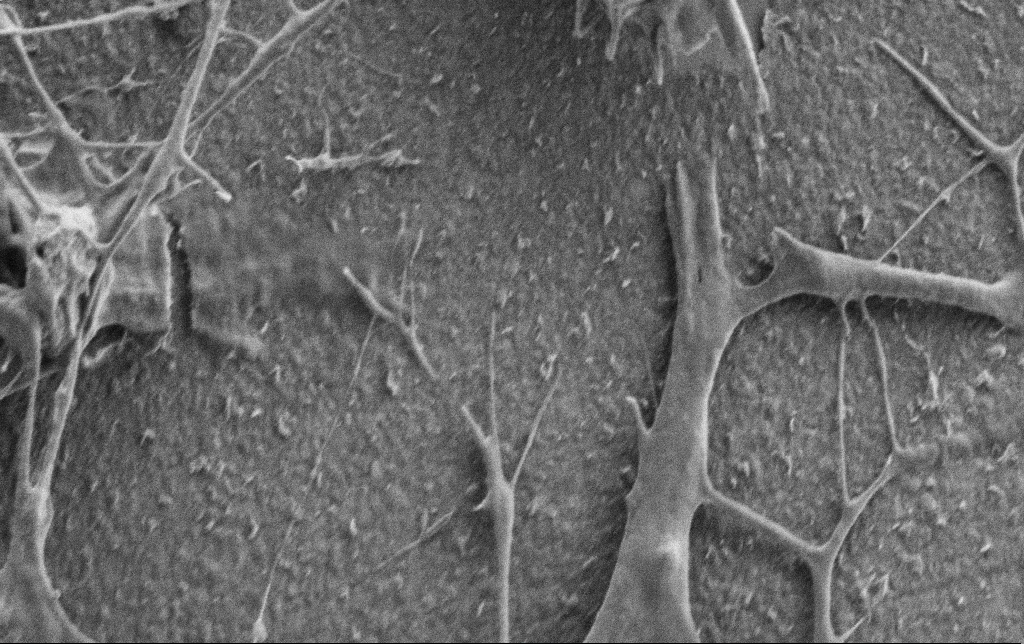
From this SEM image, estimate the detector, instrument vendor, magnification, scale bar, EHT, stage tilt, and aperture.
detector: SE2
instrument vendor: Zeiss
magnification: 20 K X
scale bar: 2000 nm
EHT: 0.9 kV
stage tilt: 0°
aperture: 30 µm